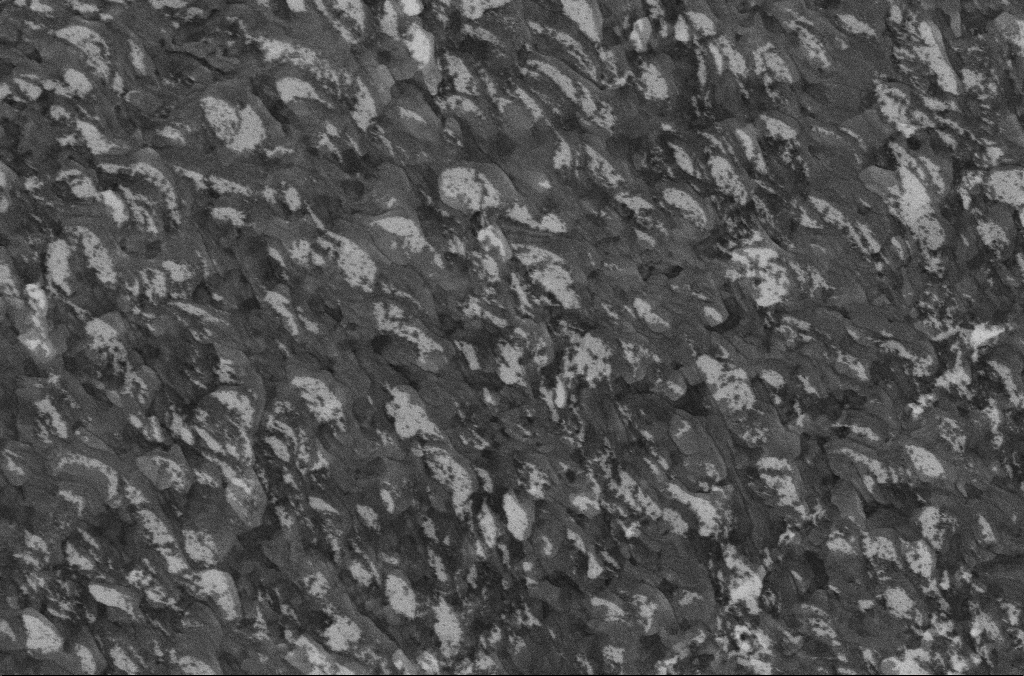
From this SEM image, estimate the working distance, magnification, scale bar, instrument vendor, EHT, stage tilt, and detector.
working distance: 6.7 mm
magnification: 20 K X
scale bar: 2000 nm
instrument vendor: Zeiss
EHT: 5 kV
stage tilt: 0°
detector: InLens